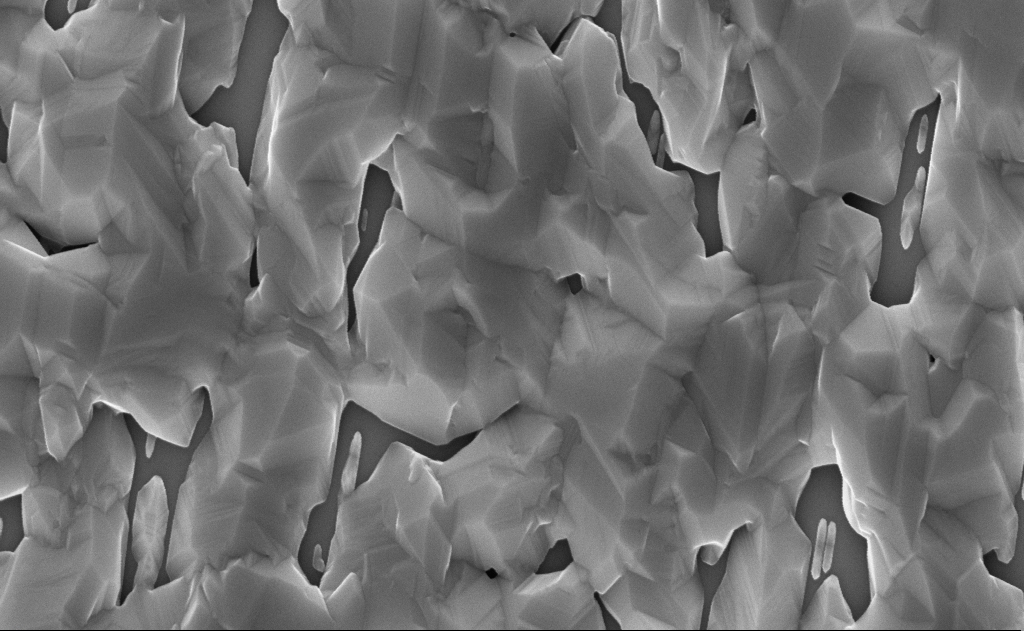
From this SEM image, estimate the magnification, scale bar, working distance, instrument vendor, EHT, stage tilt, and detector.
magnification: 40 K X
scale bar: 1000 nm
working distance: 14 mm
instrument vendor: Zeiss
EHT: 10 kV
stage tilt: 0°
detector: InLens